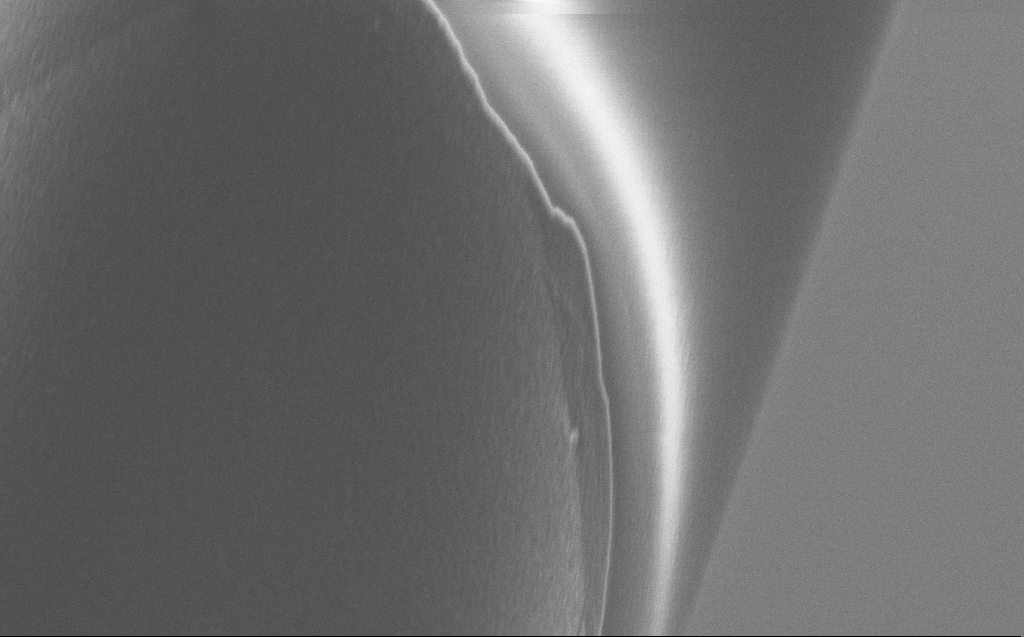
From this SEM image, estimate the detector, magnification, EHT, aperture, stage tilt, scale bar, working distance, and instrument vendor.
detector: InLens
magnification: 13.55 K X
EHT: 5 kV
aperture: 30 µm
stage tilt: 45°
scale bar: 2000 nm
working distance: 6 mm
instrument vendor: Zeiss